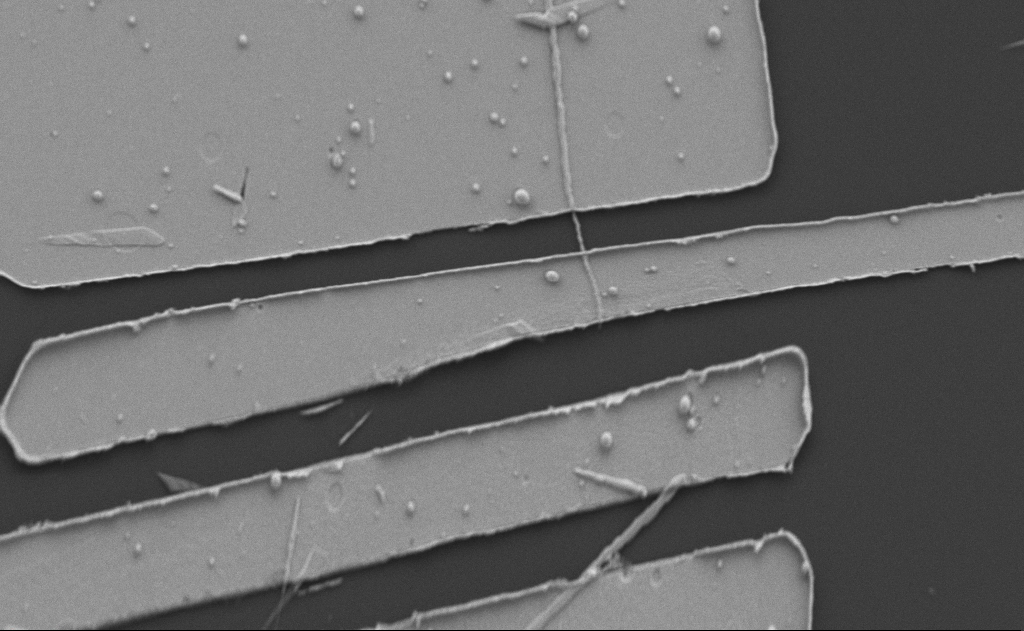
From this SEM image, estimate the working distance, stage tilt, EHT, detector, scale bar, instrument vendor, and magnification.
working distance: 10 mm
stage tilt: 0°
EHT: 5 kV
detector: SE2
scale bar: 2000 nm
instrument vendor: Zeiss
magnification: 10.08 K X